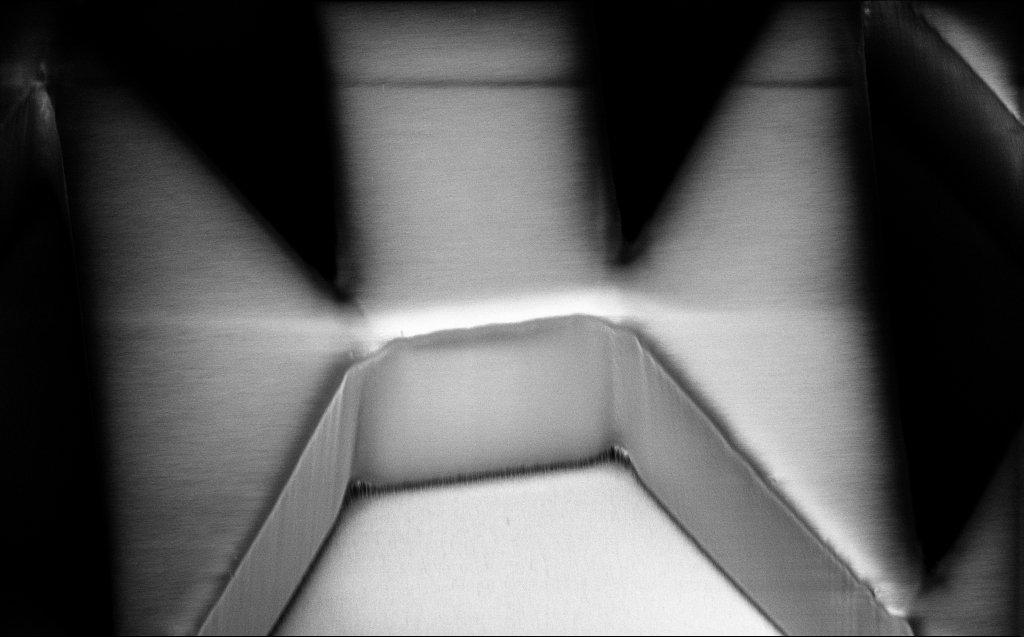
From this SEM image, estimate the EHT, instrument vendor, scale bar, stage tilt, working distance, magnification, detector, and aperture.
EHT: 1 kV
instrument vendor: Zeiss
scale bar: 20000 nm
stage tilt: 45°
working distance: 6 mm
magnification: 2.72 K X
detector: InLens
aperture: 30 µm